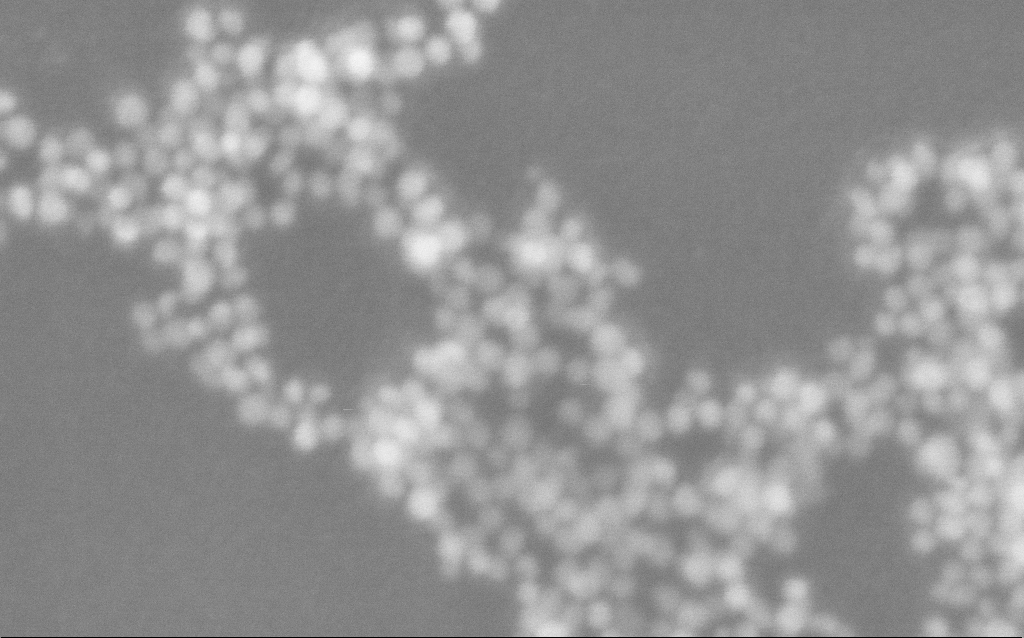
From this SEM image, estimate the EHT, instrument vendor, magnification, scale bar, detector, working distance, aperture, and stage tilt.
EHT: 10 kV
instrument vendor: Zeiss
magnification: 718.59 K X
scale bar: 100 nm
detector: InLens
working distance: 3.2 mm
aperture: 30 µm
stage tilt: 0°